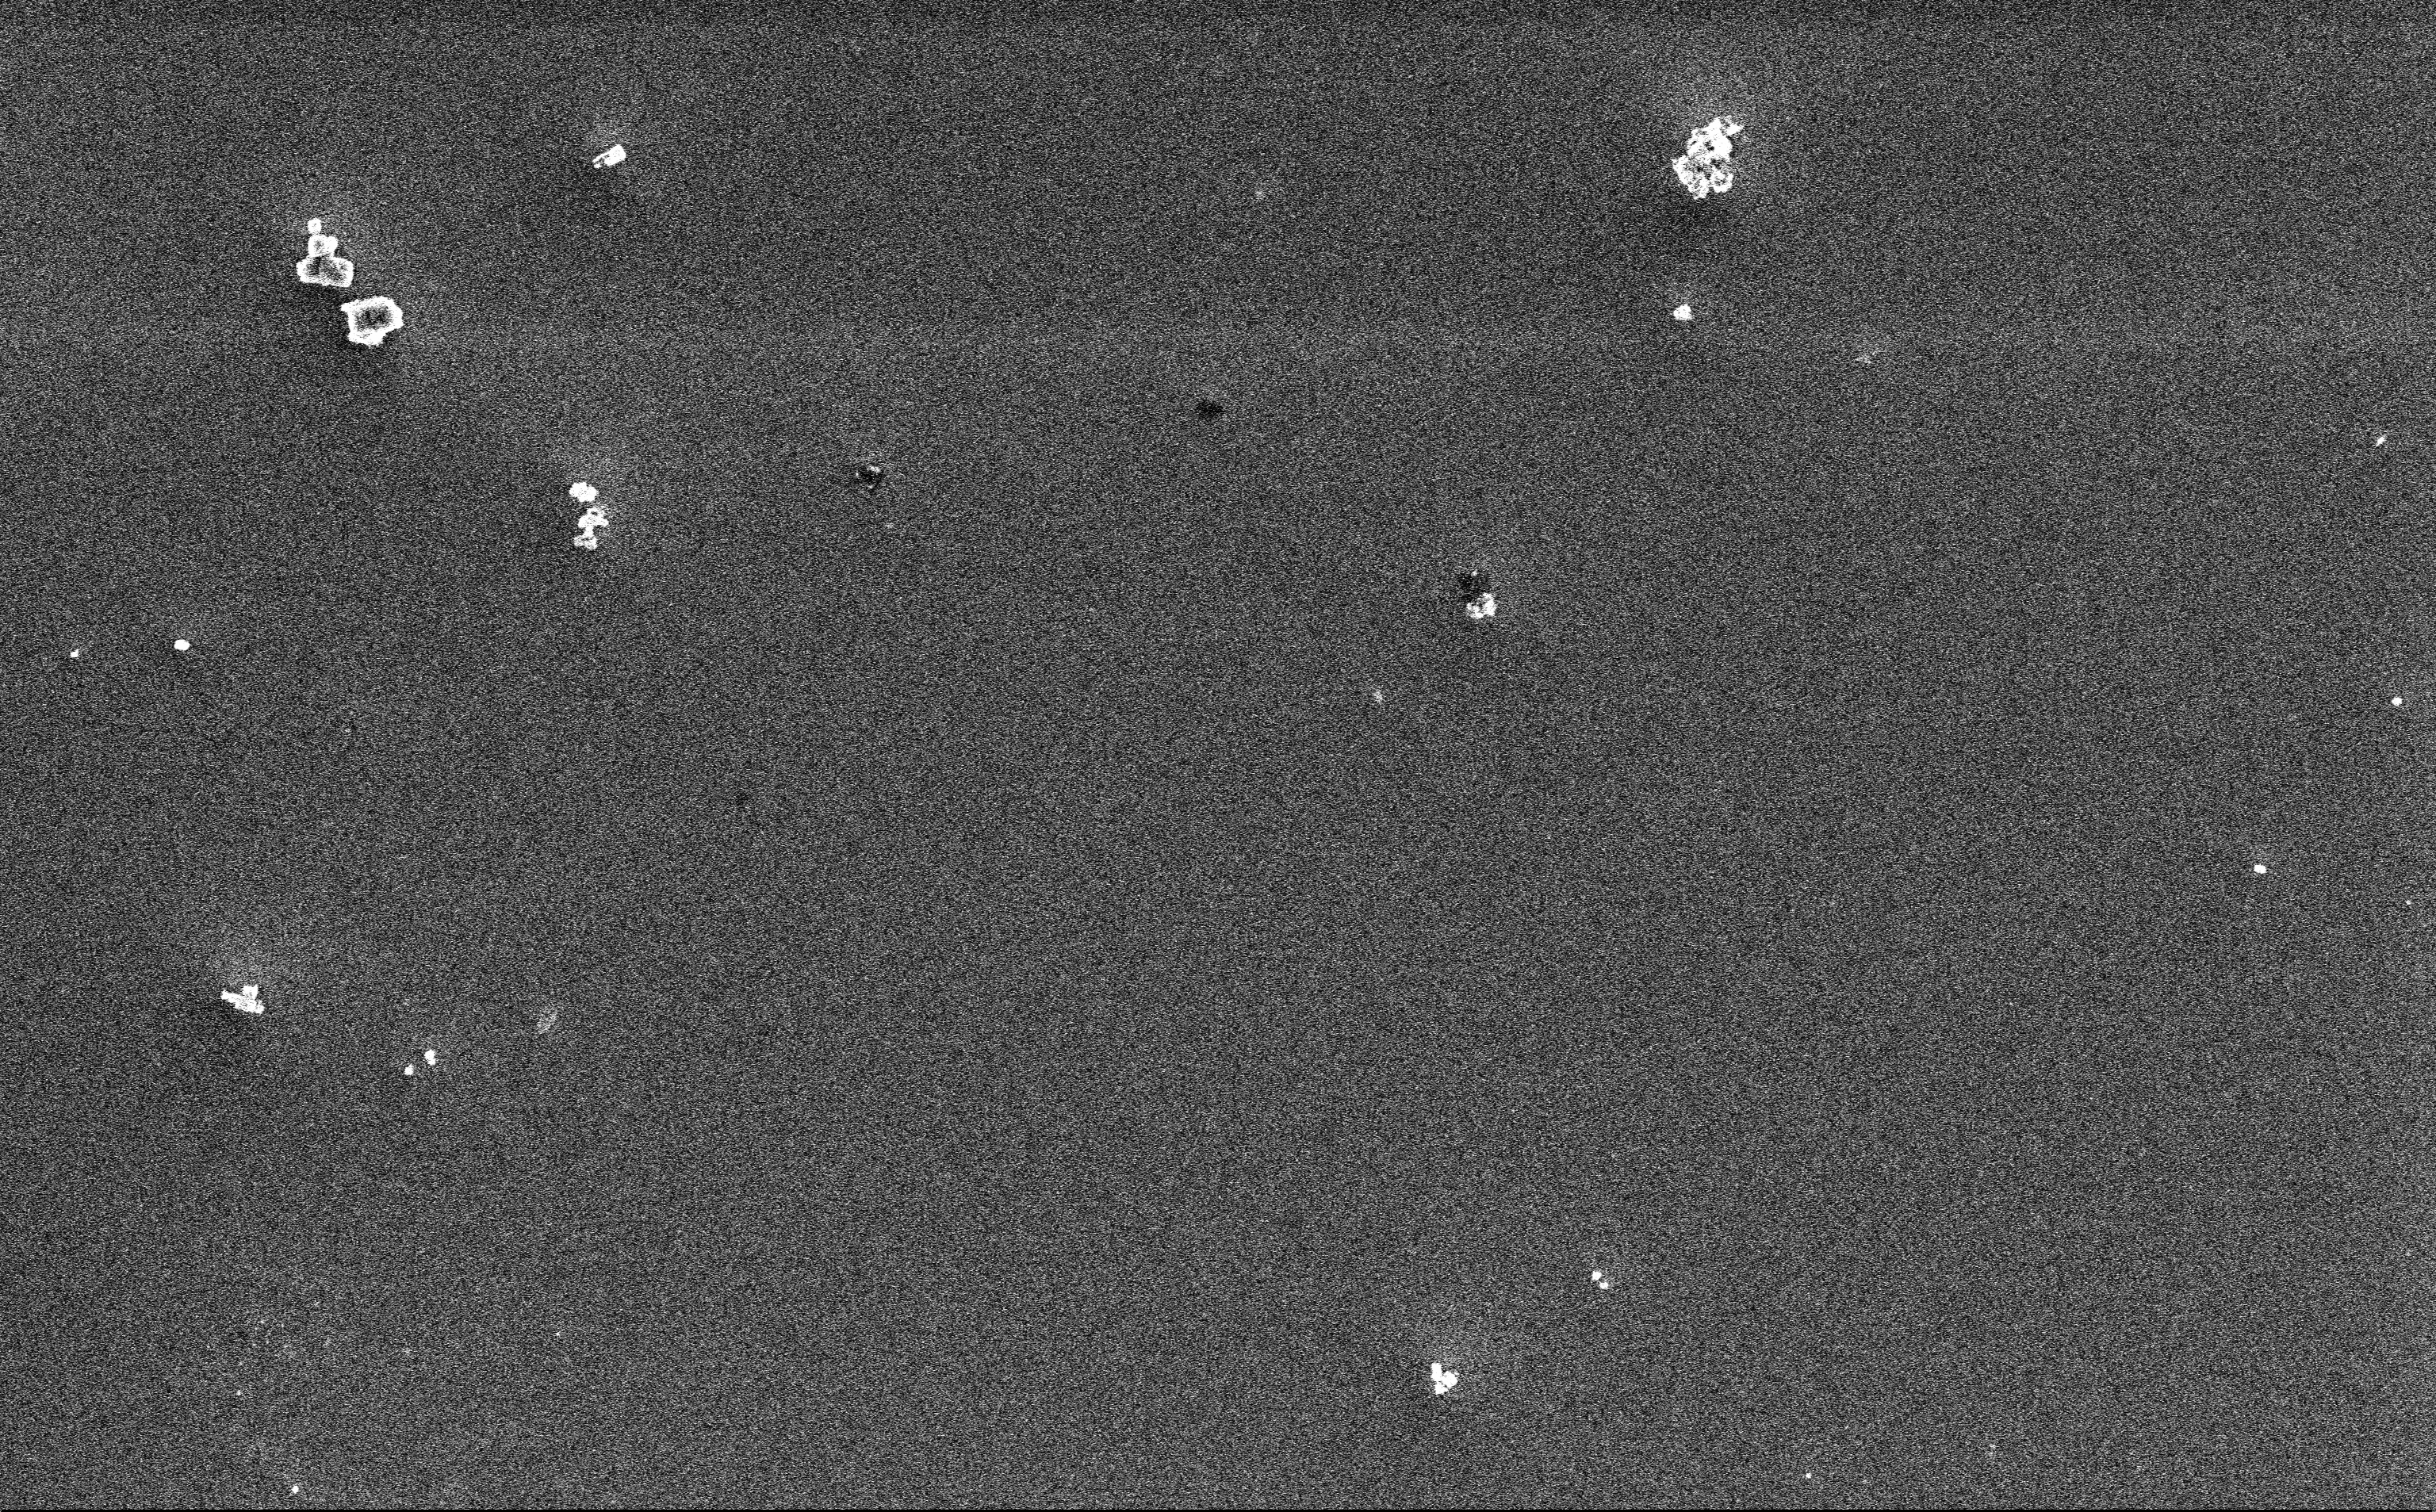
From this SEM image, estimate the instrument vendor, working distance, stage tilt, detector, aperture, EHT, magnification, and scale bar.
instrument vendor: Zeiss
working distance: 3 mm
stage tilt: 0°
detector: InLens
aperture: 30 µm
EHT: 3 kV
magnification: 12.85 K X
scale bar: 2000 nm